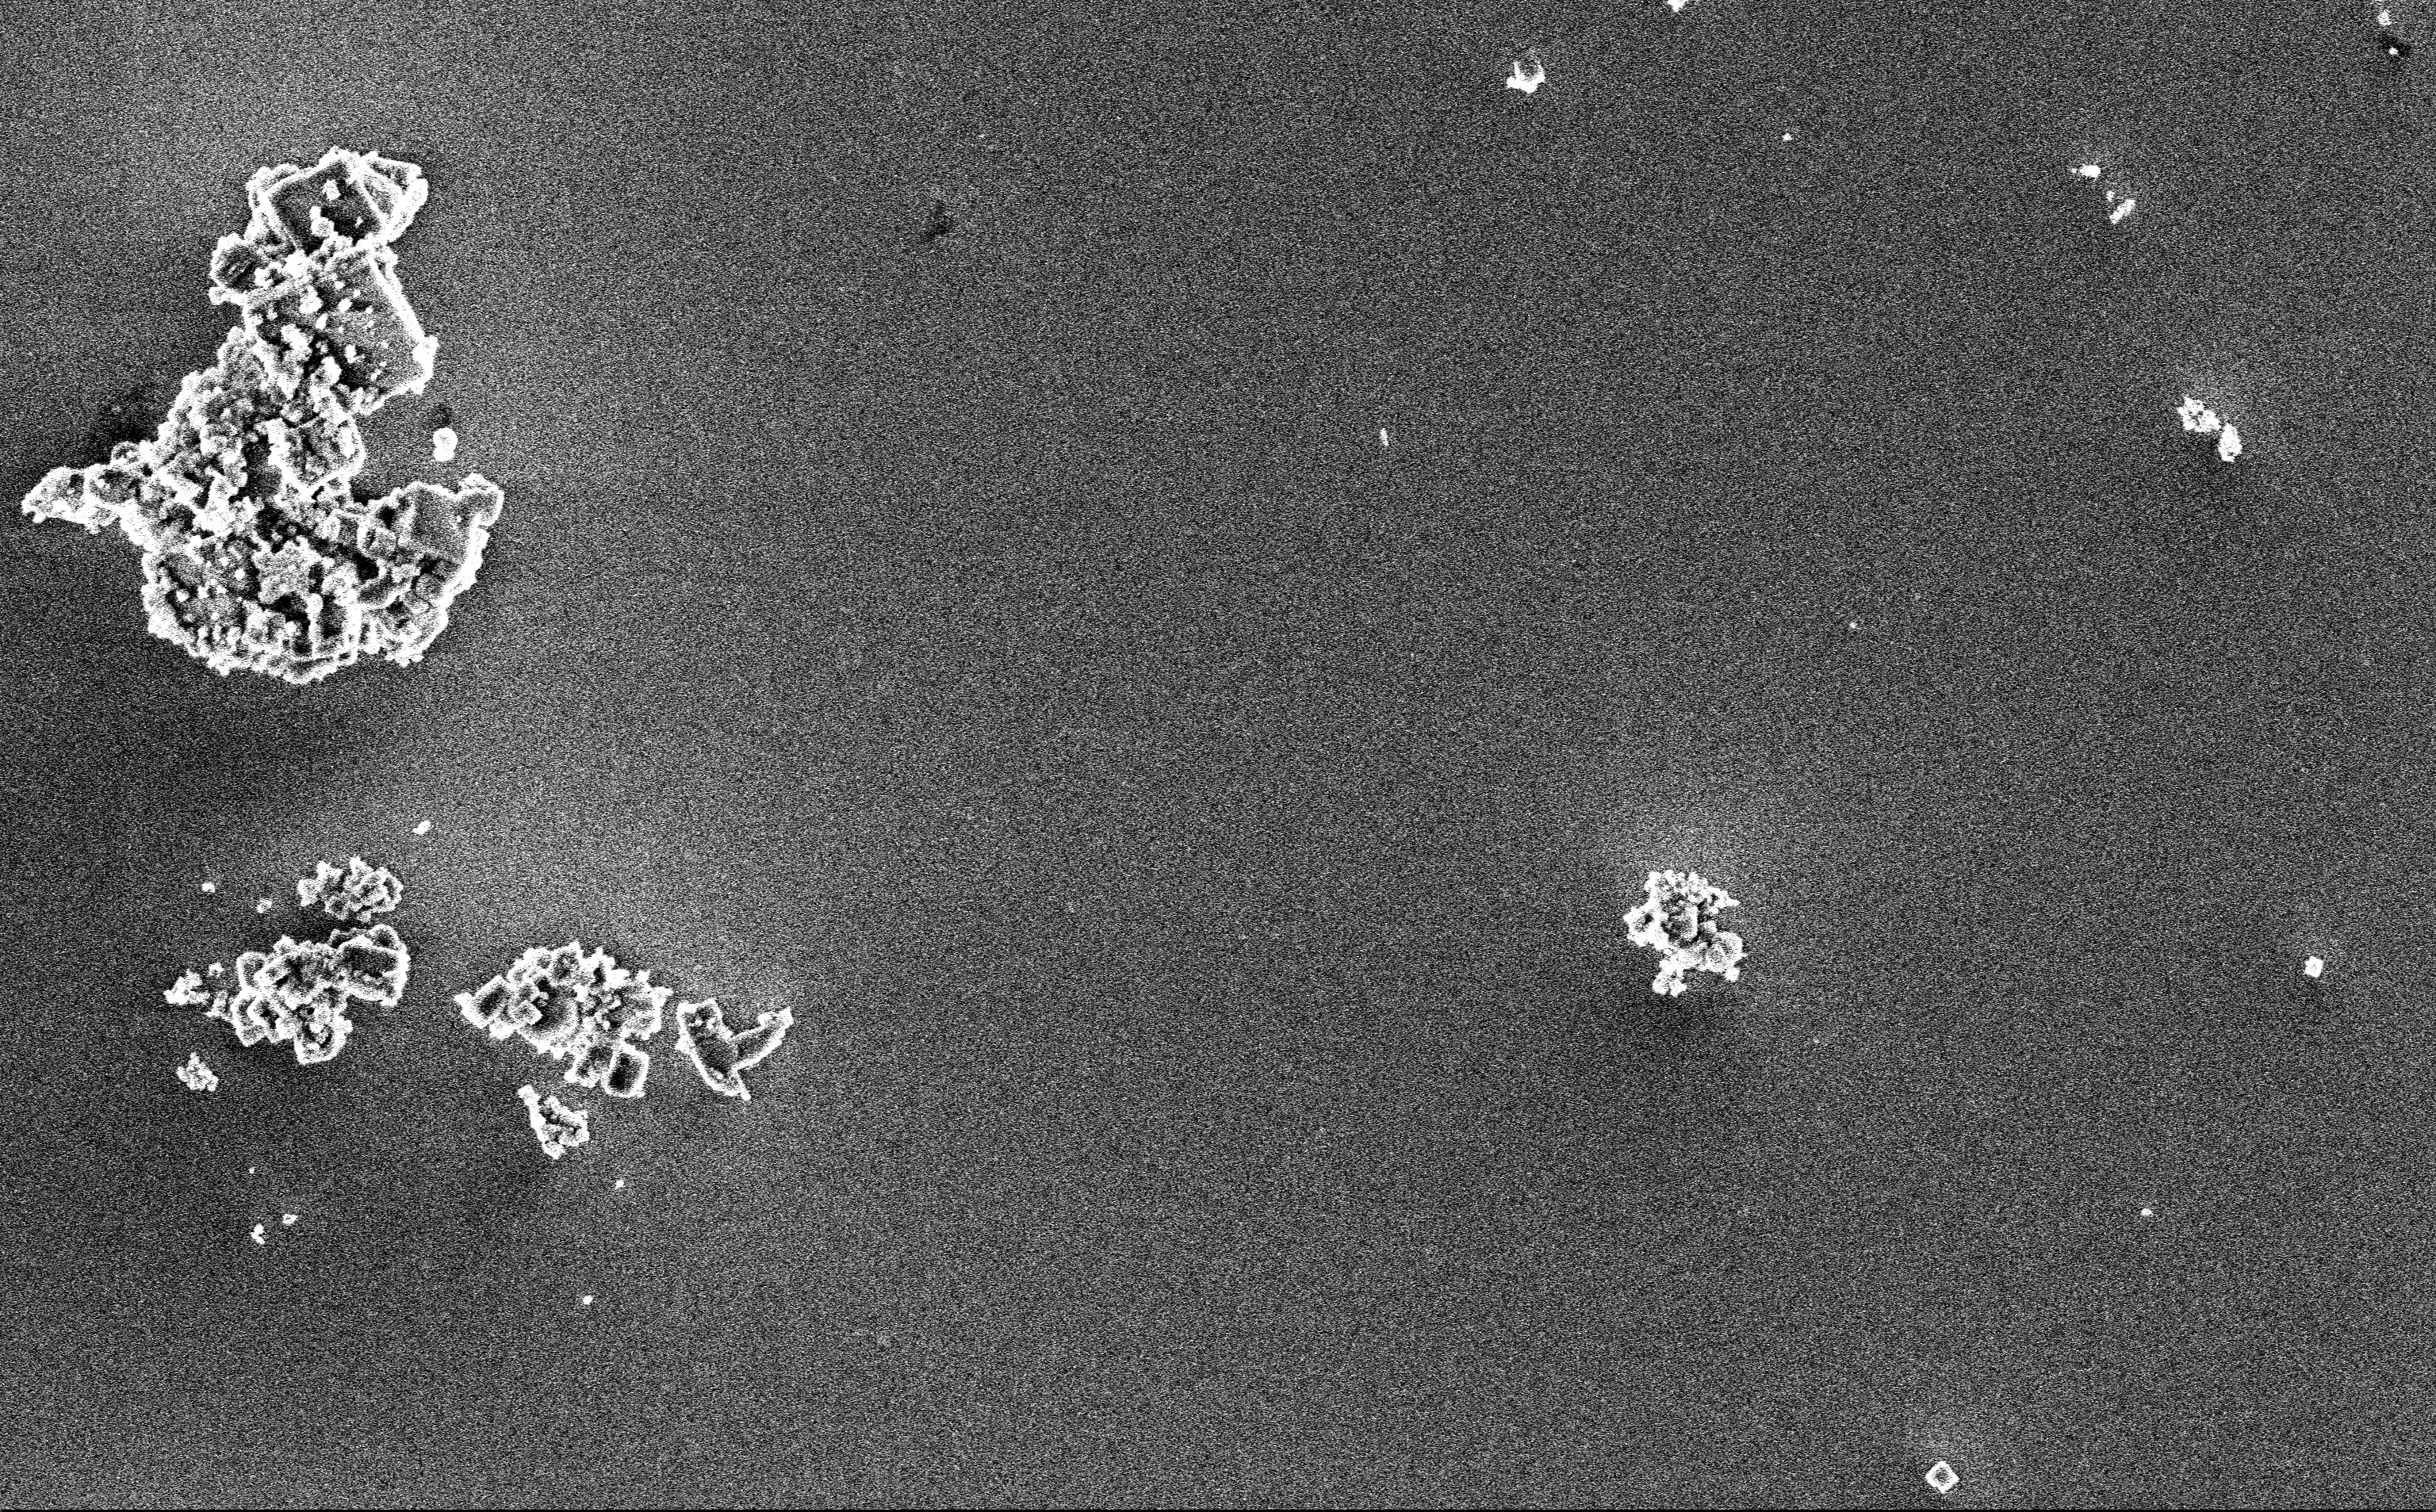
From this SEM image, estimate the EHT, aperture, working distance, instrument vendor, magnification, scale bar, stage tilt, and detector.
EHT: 3 kV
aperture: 30 µm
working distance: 3 mm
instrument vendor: Zeiss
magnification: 12.85 K X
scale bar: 2000 nm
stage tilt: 0°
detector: InLens